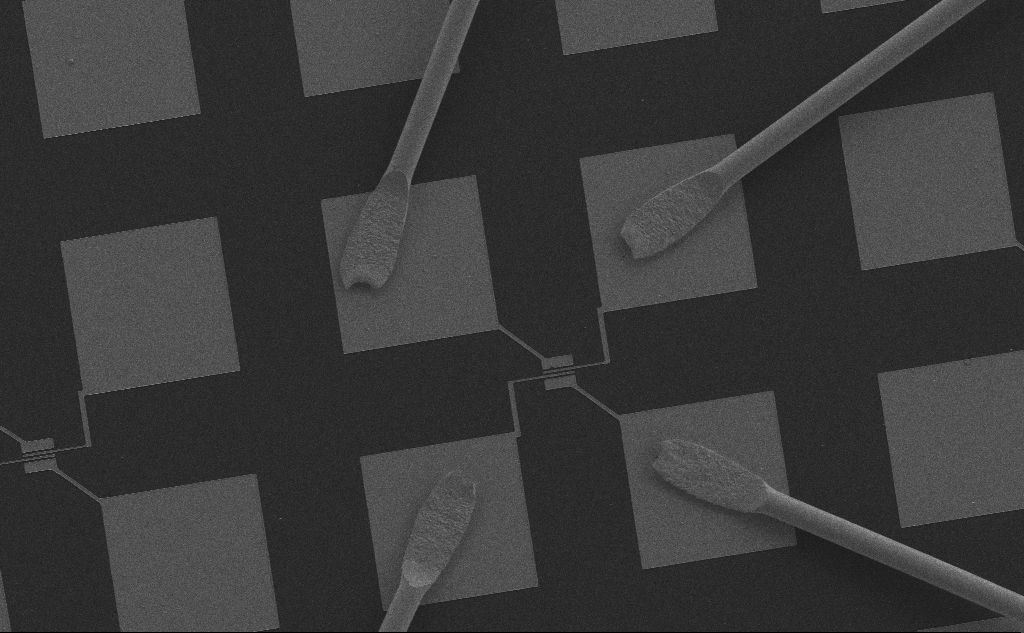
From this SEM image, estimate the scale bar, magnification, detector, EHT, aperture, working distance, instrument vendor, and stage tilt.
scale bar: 100000 nm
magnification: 0.385 K X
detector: SE2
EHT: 10 kV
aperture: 30 µm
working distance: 6 mm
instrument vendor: Zeiss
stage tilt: -0.1°